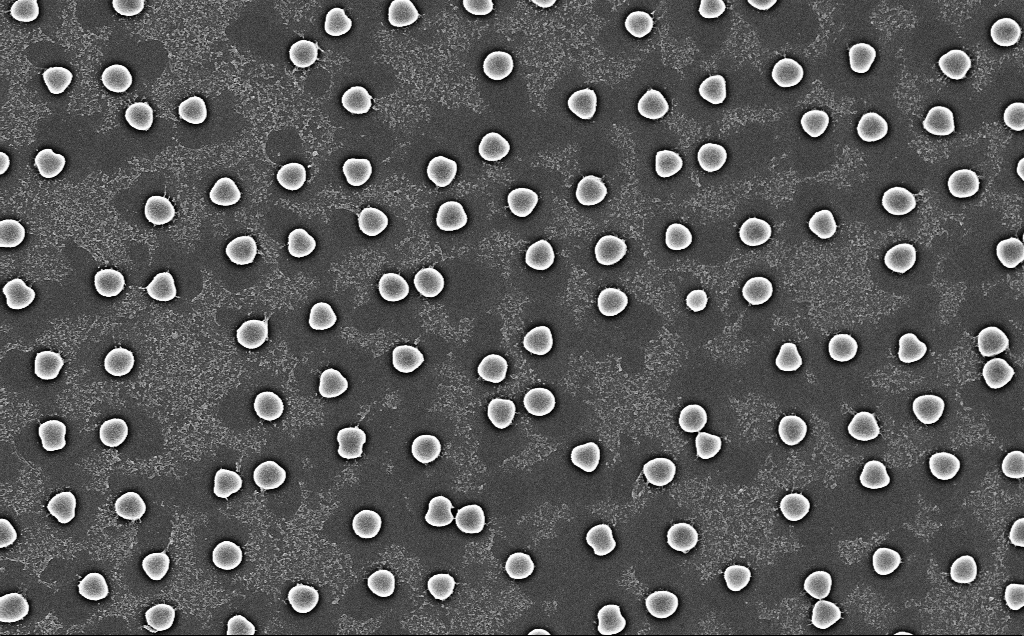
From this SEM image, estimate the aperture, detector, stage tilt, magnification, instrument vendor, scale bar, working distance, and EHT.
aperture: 30 µm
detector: InLens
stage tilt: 0°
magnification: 17.75 K X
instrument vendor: Zeiss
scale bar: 2000 nm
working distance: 7 mm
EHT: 5 kV